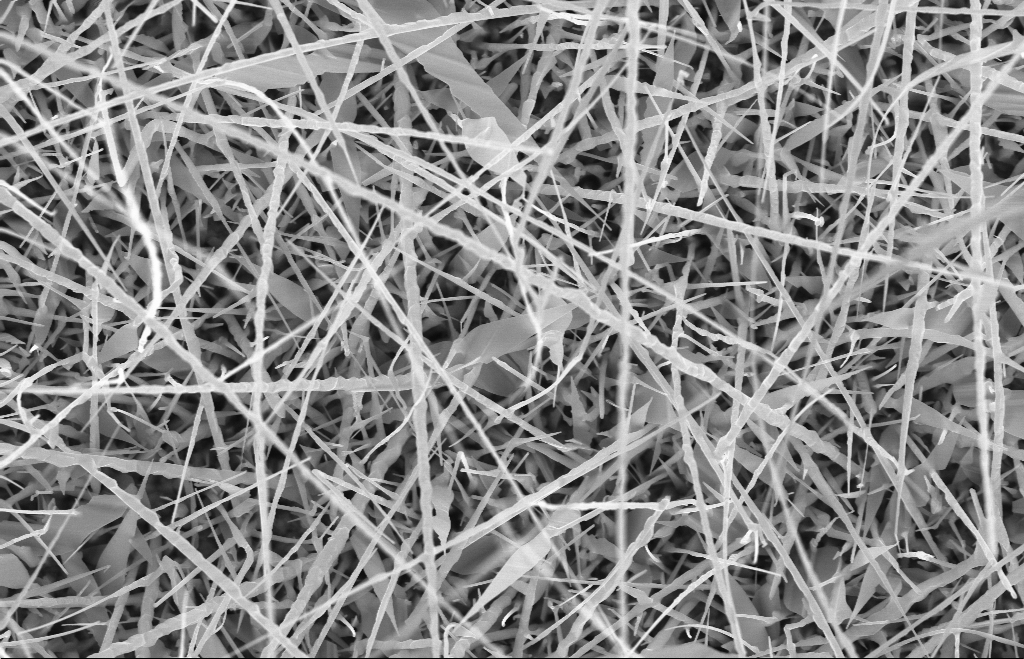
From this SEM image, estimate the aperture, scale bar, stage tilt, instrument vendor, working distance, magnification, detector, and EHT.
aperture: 30 µm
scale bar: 1000 nm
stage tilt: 0°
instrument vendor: Zeiss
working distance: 8 mm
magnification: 20 K X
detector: InLens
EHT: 10 kV